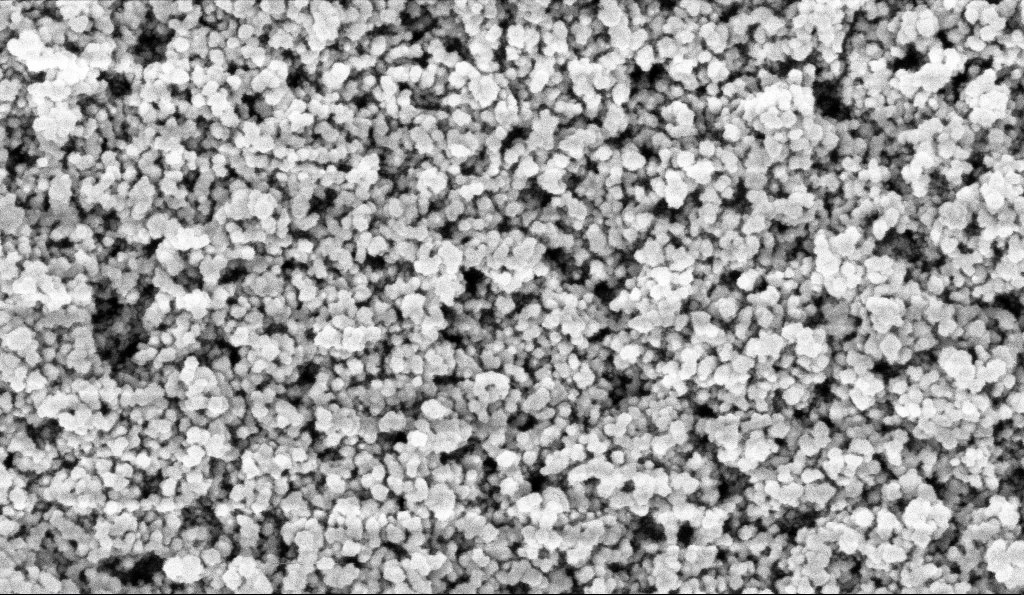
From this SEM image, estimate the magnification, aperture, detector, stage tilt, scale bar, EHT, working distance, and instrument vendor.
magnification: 135 K X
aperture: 30 µm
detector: InLens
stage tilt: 0°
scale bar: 100 nm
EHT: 5 kV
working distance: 6 mm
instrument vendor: Zeiss